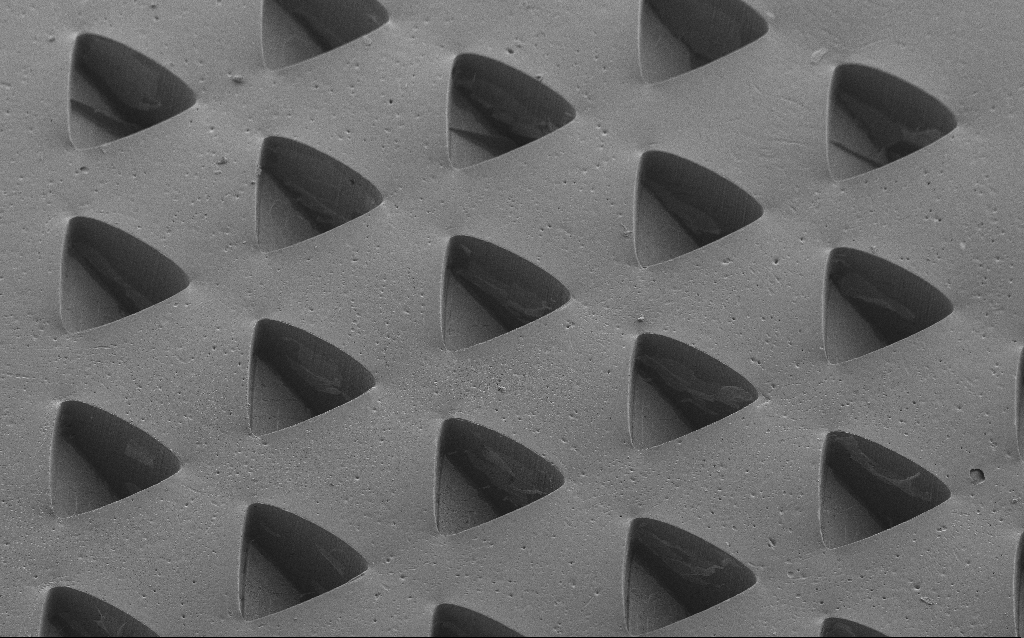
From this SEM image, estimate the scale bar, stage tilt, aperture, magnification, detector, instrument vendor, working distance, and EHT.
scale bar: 100000 nm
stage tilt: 35°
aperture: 30 µm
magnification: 0.185 K X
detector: SE2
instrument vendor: Zeiss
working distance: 8 mm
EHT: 5 kV